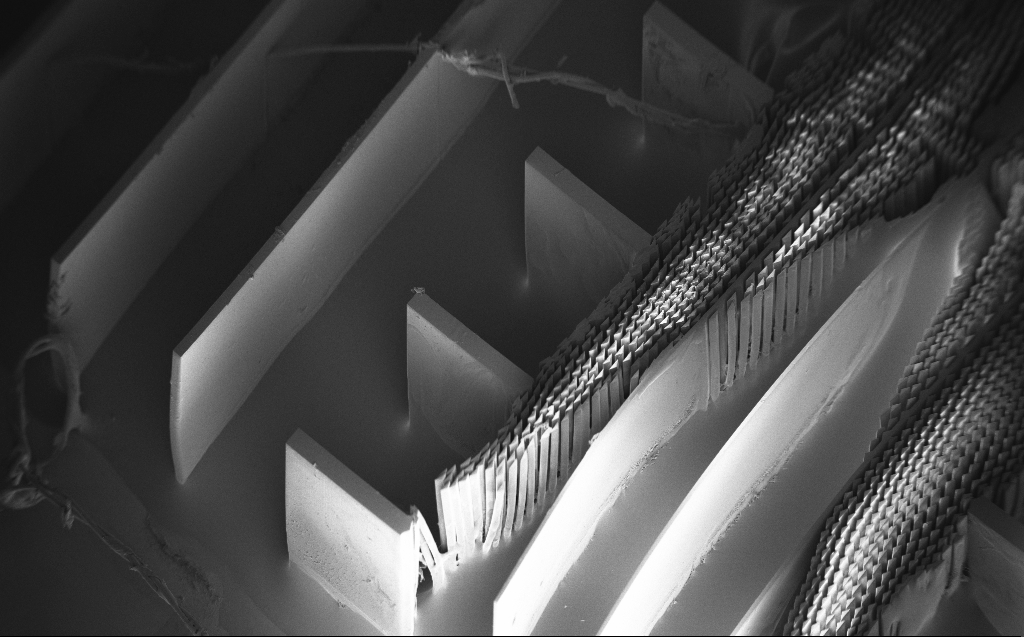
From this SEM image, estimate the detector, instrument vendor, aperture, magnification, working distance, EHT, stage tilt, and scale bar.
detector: InLens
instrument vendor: Zeiss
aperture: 30 µm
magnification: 0.124 K X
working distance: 7 mm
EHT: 3 kV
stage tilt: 45°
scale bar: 200000 nm